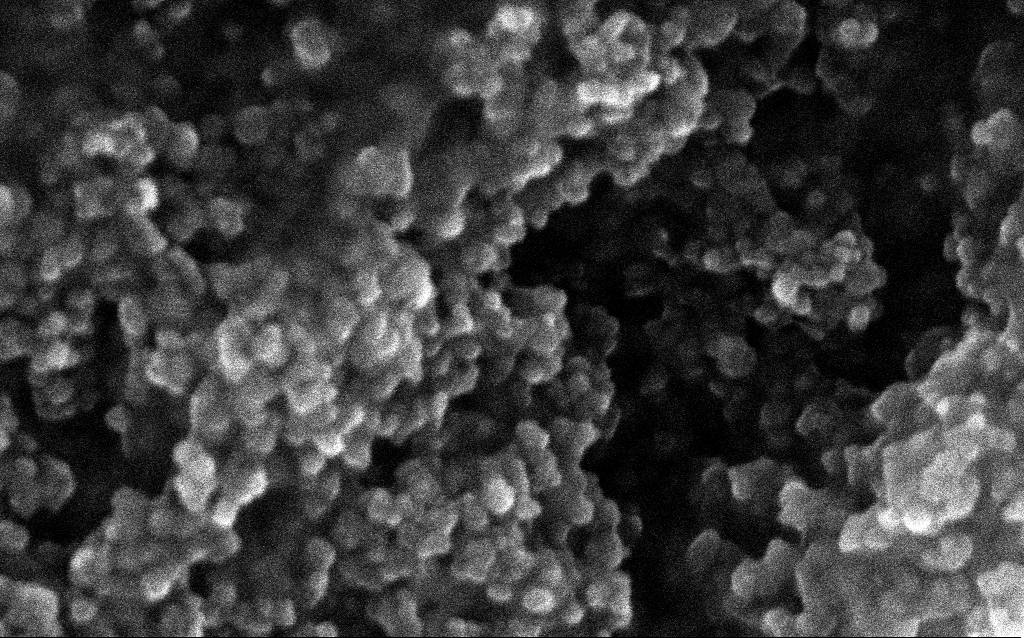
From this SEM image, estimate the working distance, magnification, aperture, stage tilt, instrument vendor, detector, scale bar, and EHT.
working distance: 2.6 mm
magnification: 348.1 K X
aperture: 30 µm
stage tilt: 0°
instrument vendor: Zeiss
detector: InLens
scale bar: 100 nm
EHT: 10 kV